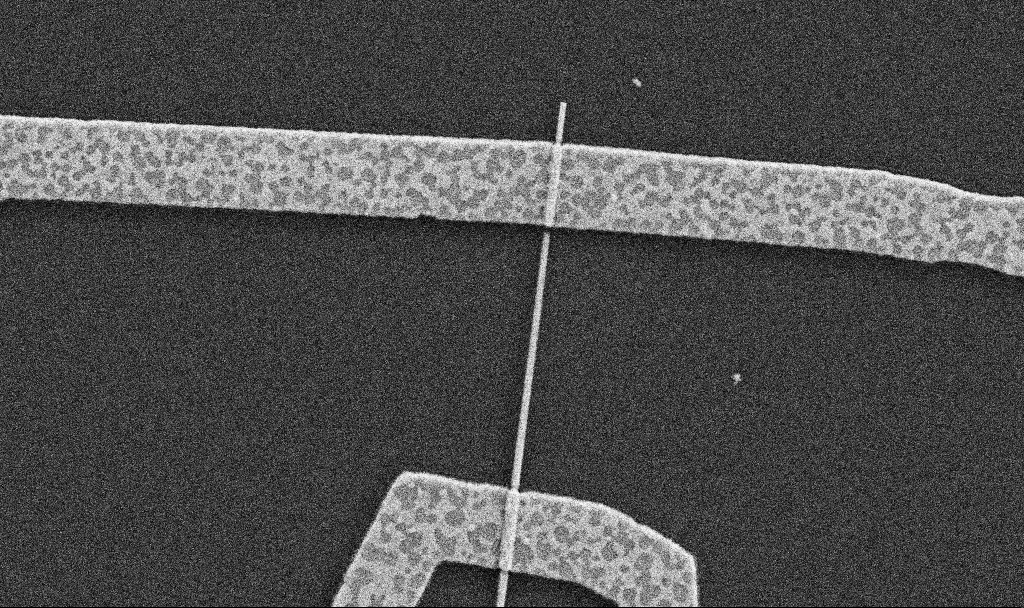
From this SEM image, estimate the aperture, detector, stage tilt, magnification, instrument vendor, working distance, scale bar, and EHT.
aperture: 30 µm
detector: SE2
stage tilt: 0°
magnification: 30 K X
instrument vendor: Zeiss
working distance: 8.7 mm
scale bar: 1000 nm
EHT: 5 kV